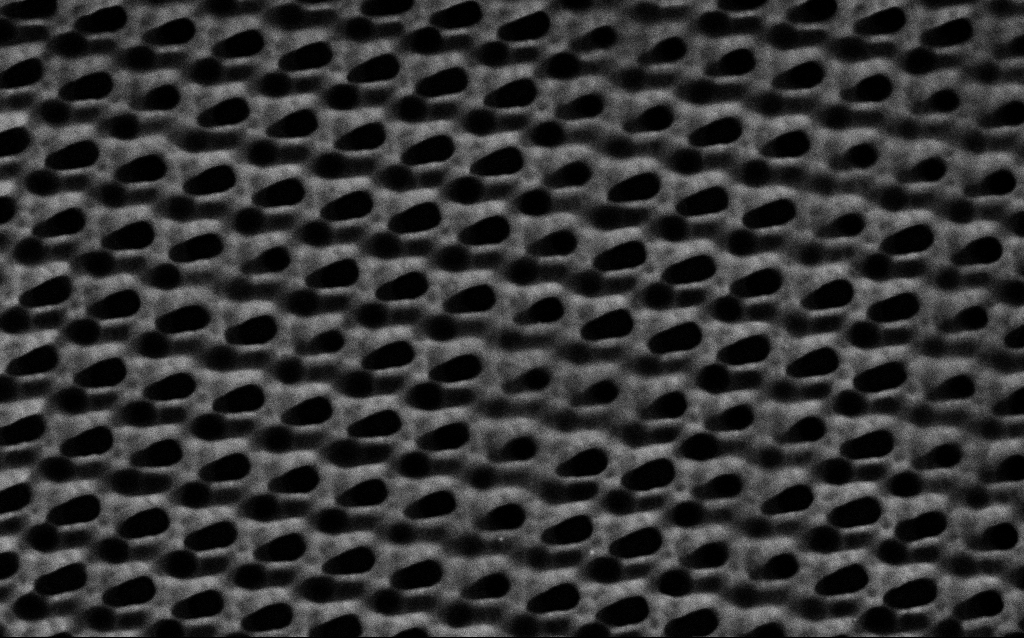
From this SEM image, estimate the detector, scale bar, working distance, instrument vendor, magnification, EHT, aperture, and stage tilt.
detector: SE2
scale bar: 1000 nm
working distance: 4.6 mm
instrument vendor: Zeiss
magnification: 55.57 K X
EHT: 3 kV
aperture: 30 µm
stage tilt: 0°